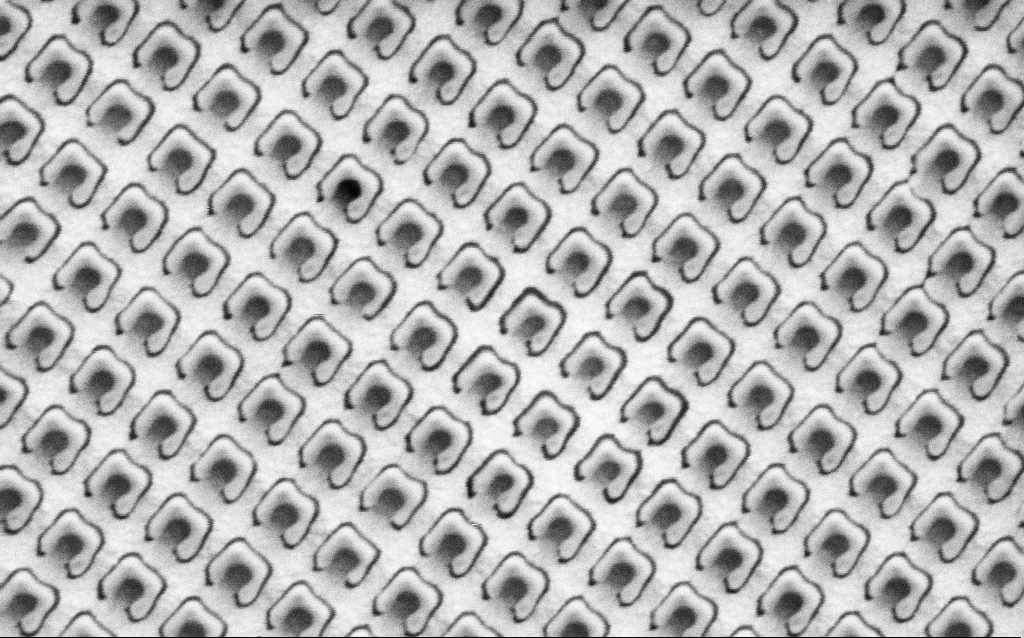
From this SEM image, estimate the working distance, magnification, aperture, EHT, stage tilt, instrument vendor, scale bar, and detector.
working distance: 8 mm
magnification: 60.31 K X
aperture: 30 µm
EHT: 1.5 kV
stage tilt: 0°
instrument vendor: Zeiss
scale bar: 1000 nm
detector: SE2